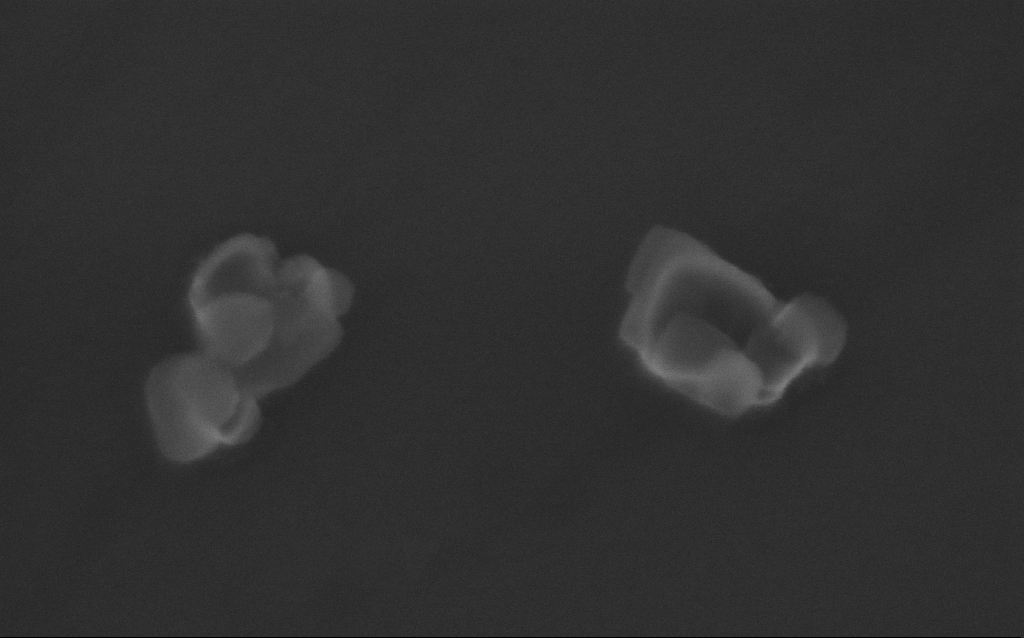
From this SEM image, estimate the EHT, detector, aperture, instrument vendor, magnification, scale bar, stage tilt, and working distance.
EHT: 10 kV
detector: InLens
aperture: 30 µm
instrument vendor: Zeiss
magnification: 343.86 K X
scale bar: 200 nm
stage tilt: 0°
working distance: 4 mm